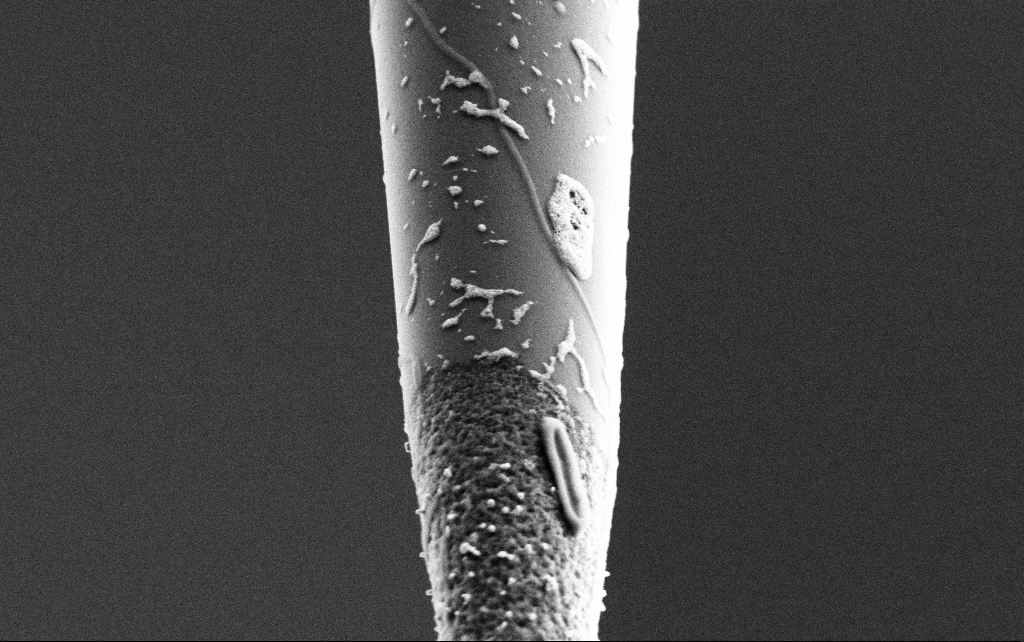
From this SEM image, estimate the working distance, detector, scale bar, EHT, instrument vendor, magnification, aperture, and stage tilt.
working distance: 7.7 mm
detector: SE2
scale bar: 1000 nm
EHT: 3 kV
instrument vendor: Zeiss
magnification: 25 K X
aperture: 30 µm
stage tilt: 45°